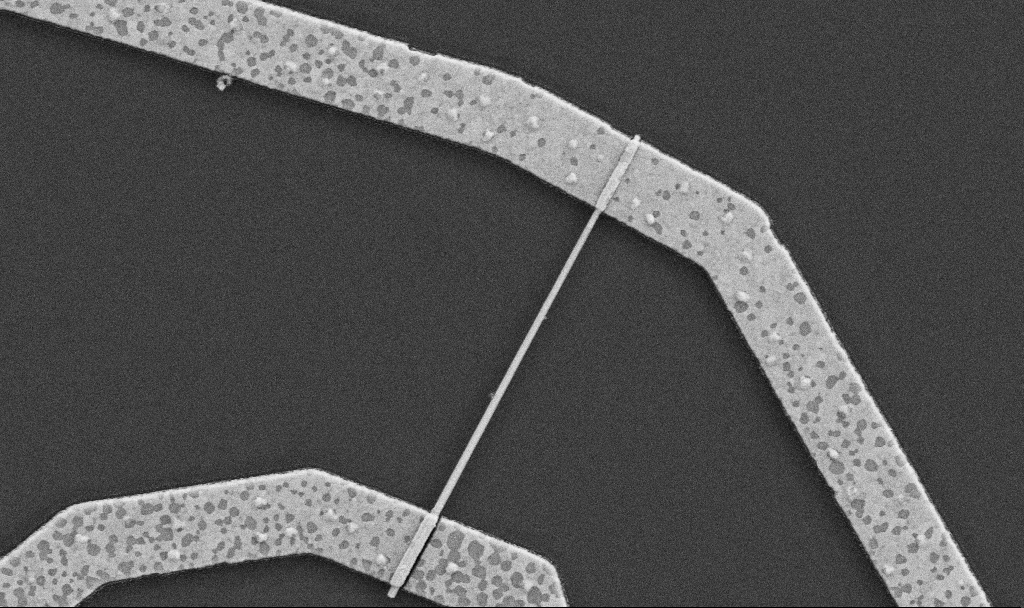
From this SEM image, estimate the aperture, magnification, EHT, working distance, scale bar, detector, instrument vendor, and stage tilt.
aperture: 30 µm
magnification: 30 K X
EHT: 5 kV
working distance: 8.7 mm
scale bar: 1000 nm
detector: SE2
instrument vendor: Zeiss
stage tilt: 0°